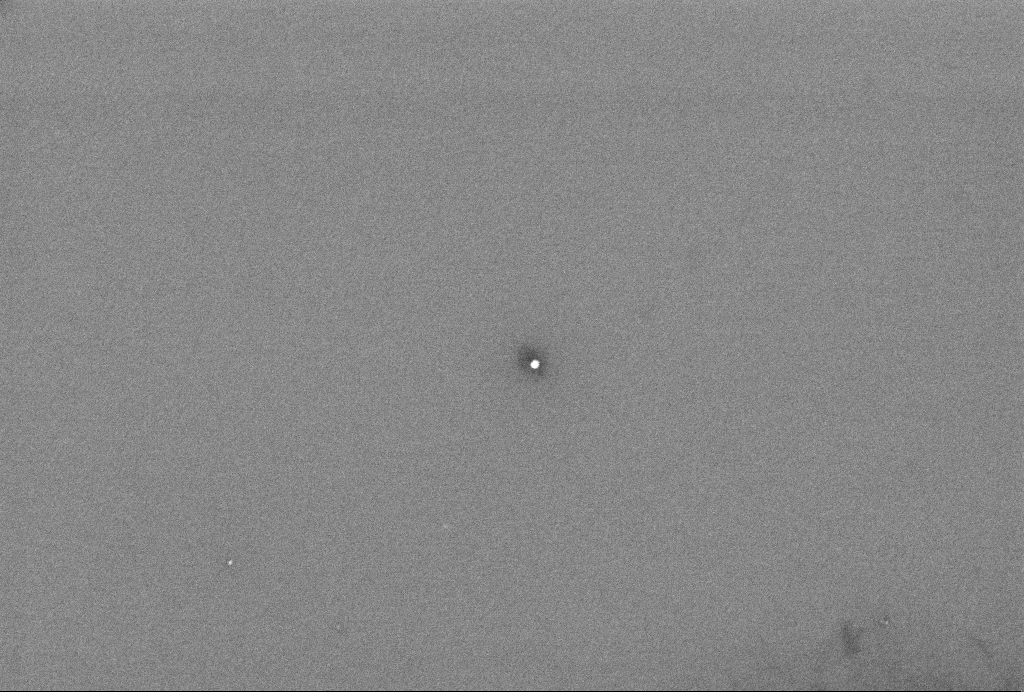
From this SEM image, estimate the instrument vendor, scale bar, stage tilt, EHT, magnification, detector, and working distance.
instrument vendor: Zeiss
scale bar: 200 nm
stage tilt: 0°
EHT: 2 kV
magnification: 107.34 K X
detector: InLens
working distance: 3.3 mm